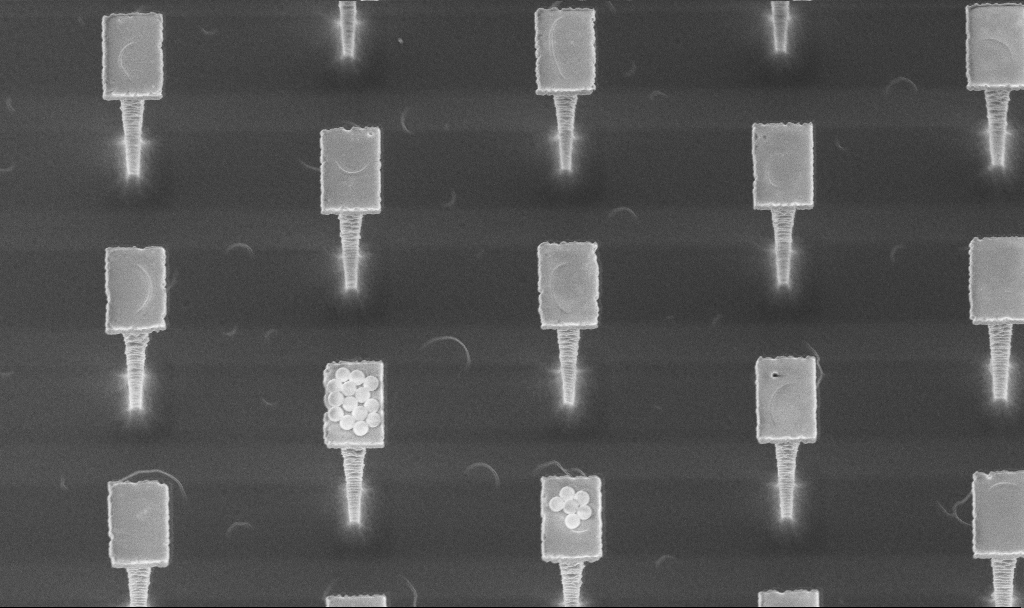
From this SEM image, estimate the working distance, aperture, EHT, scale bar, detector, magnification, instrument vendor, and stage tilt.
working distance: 4.5 mm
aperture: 30 µm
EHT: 5 kV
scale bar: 2000 nm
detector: InLens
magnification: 7.62 K X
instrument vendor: Zeiss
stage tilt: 20°